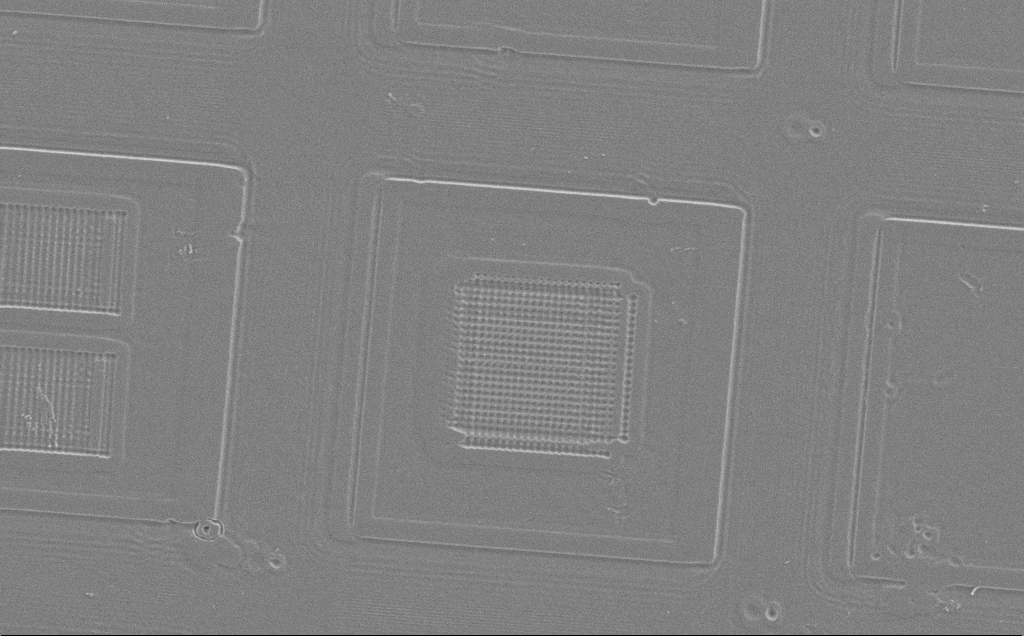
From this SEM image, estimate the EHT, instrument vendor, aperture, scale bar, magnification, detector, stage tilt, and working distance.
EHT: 5 kV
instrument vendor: Zeiss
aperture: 30 µm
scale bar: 20000 nm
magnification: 1.3 K X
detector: SE2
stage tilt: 0°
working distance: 10 mm